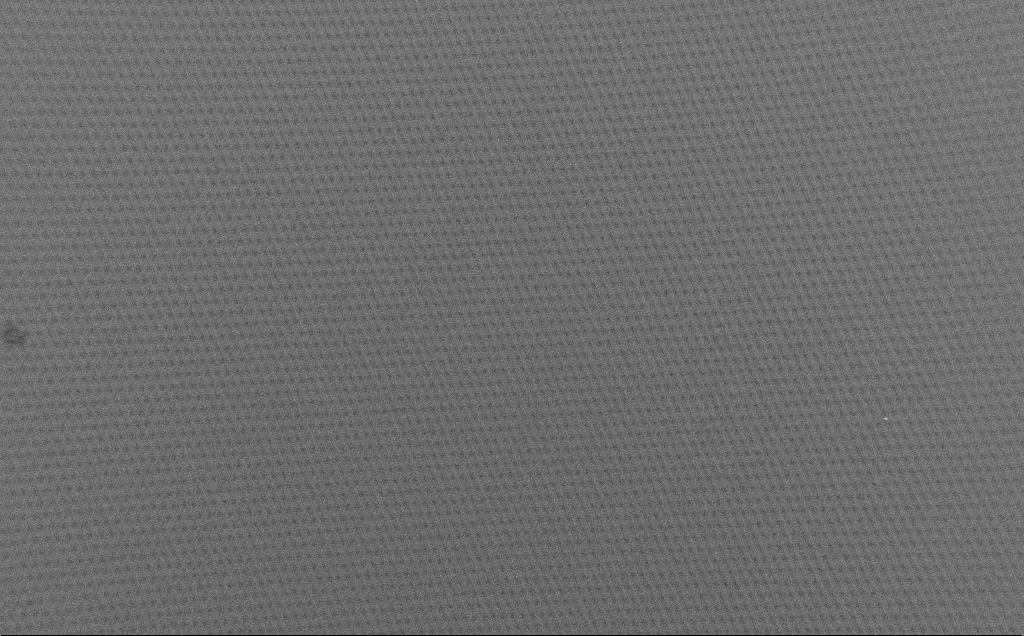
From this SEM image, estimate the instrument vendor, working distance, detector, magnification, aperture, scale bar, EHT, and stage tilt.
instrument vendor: Zeiss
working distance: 4 mm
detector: SE2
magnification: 0.176 K X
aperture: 30 µm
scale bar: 100000 nm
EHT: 1.5 kV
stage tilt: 0°